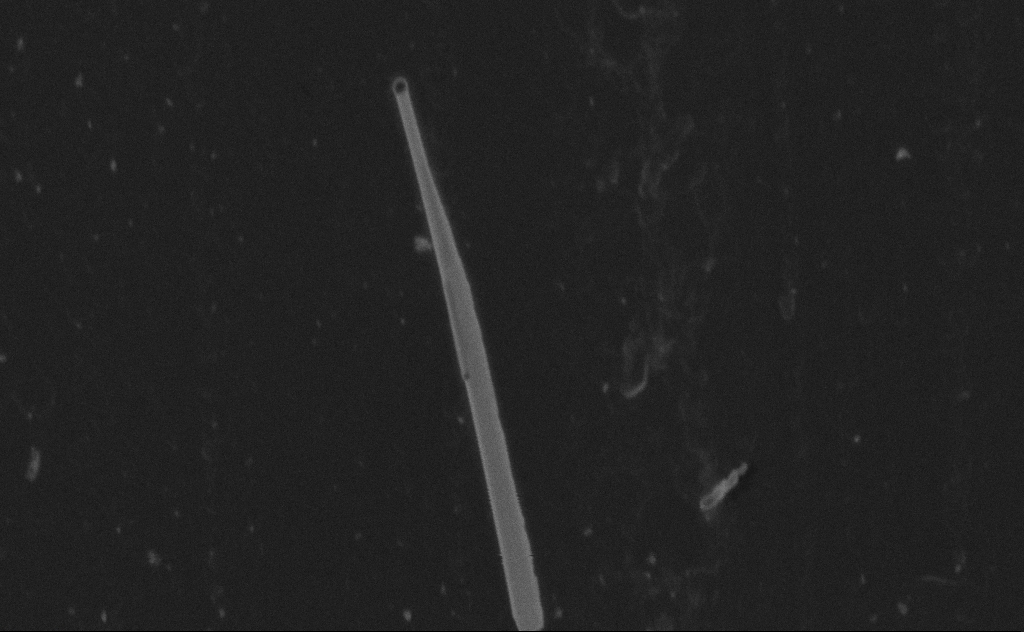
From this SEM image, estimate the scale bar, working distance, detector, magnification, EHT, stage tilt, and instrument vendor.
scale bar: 1000 nm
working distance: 9 mm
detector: SE2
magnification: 63.15 K X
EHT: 20 kV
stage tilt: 0°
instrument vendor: Zeiss